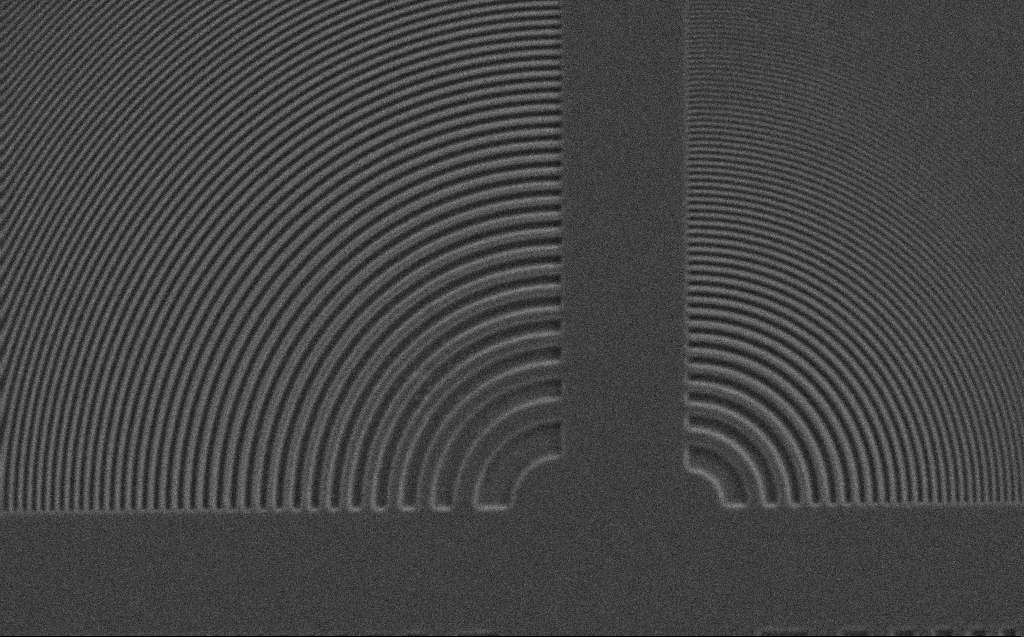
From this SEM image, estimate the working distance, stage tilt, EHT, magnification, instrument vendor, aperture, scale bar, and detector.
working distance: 3 mm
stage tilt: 0°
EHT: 5 kV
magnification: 4.67 K X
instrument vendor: Zeiss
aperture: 30 µm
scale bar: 10000 nm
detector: SE2